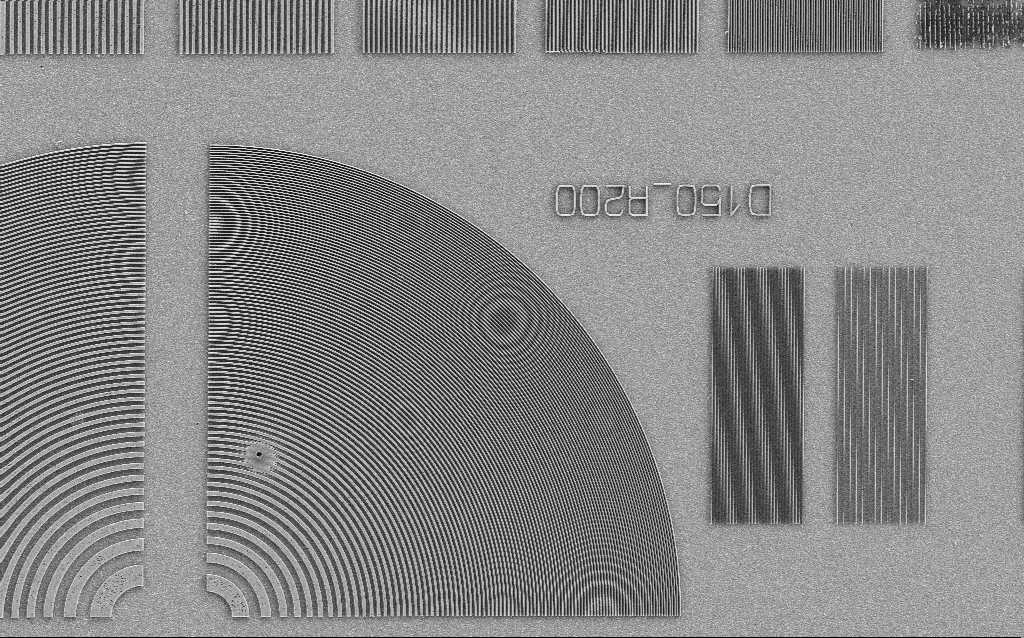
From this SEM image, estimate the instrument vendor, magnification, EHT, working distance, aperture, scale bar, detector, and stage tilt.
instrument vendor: Zeiss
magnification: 2.34 K X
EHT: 3 kV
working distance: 5 mm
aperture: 30 µm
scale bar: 10000 nm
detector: SE2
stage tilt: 0°